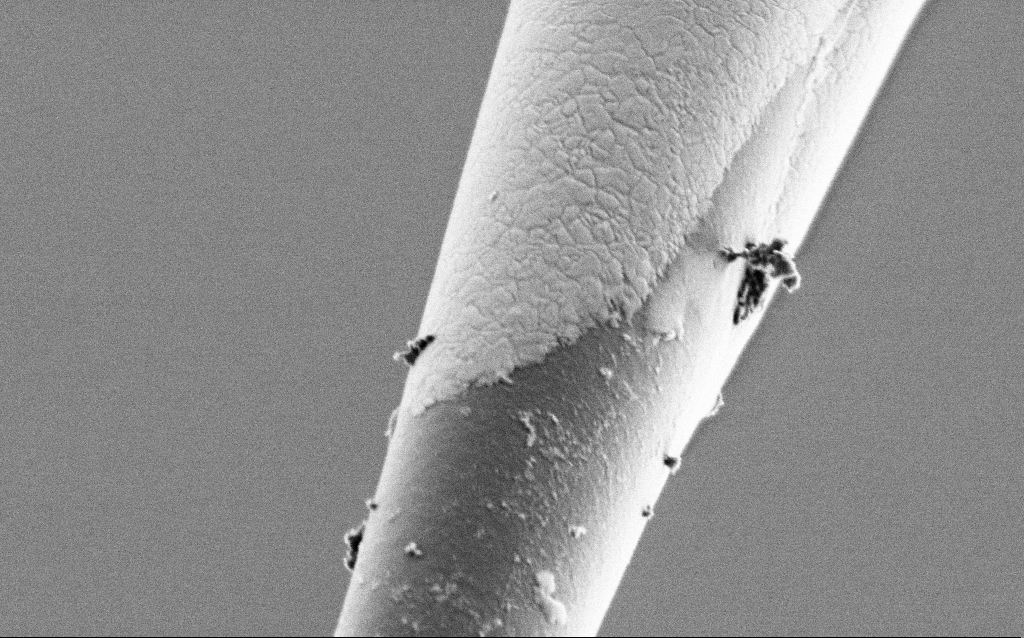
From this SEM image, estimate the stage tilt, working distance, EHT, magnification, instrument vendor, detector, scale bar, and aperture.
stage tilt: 45°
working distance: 6.5 mm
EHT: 1 kV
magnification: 50 K X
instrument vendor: Zeiss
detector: SE2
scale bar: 1000 nm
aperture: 30 µm